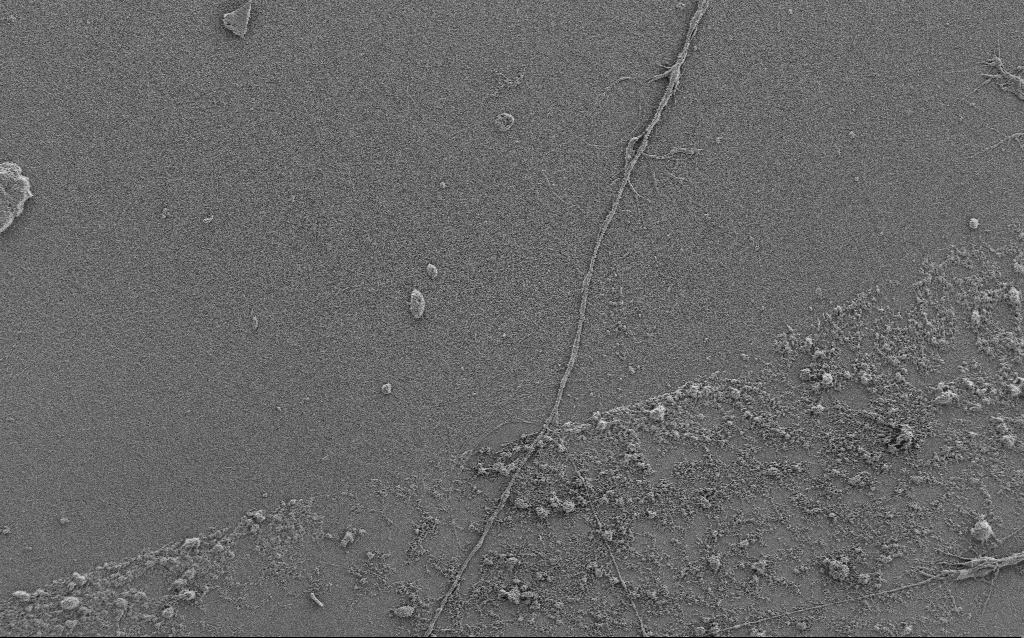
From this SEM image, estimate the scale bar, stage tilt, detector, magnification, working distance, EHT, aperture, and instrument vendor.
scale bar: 10000 nm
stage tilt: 0°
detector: SE2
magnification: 2.5 K X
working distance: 4 mm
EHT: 0.9 kV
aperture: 30 µm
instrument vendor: Zeiss